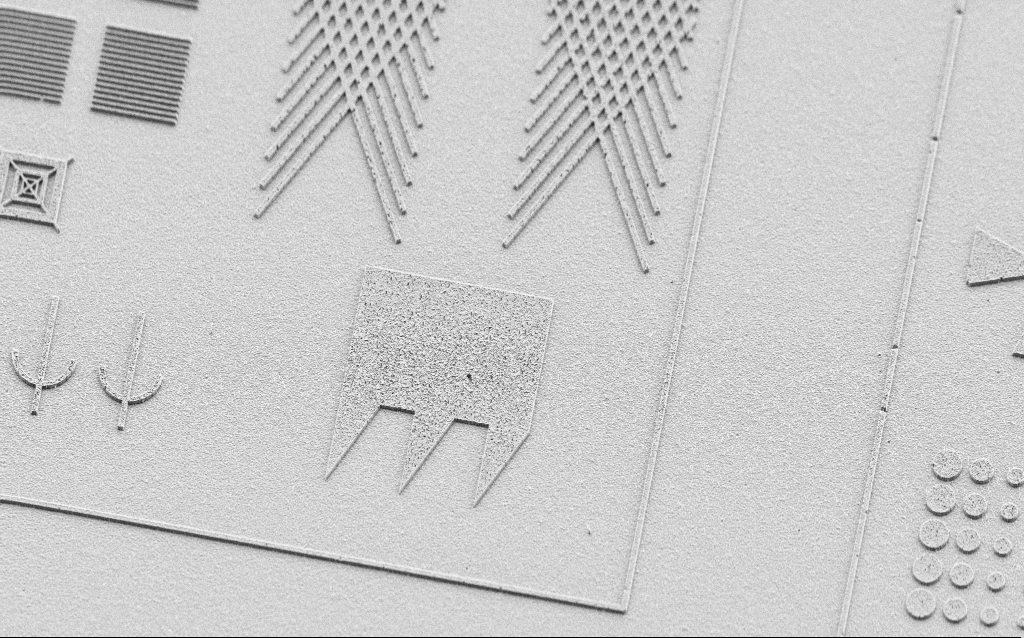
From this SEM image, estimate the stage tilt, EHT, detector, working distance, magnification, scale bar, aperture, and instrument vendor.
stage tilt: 45°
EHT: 2 kV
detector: SE2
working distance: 7 mm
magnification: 2.14 K X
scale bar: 10000 nm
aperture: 30 µm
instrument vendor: Zeiss